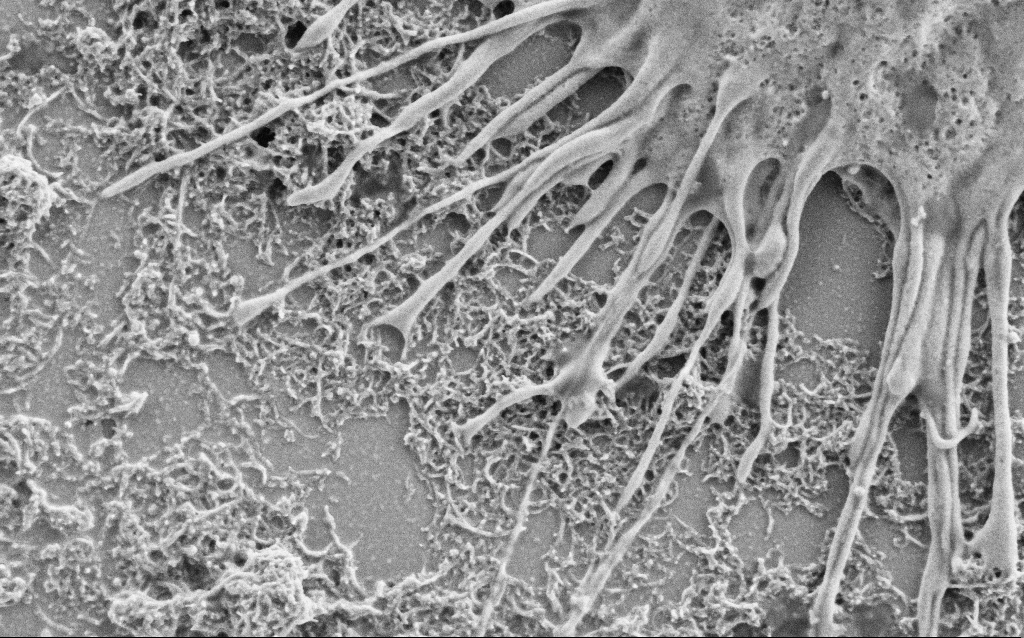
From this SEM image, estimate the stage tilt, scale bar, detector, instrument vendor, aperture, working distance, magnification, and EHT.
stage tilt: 0°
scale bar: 1000 nm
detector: SE2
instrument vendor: Zeiss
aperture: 30 µm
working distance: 6.9 mm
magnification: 25 K X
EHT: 2 kV